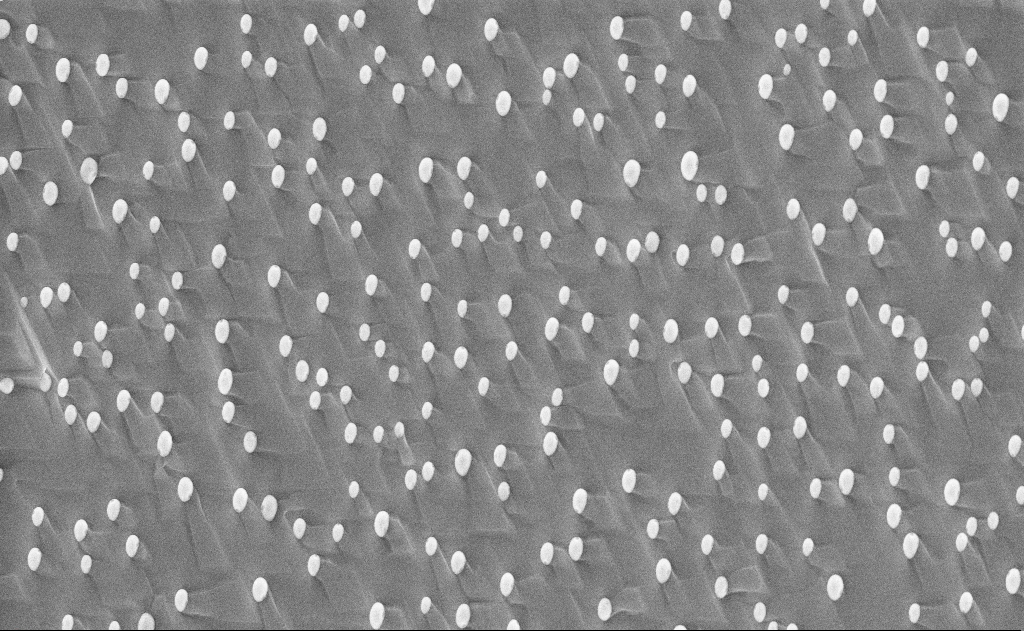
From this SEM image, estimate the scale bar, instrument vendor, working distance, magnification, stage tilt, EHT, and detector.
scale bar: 2000 nm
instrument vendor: Zeiss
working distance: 12 mm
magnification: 10 K X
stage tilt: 0°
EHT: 10 kV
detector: InLens